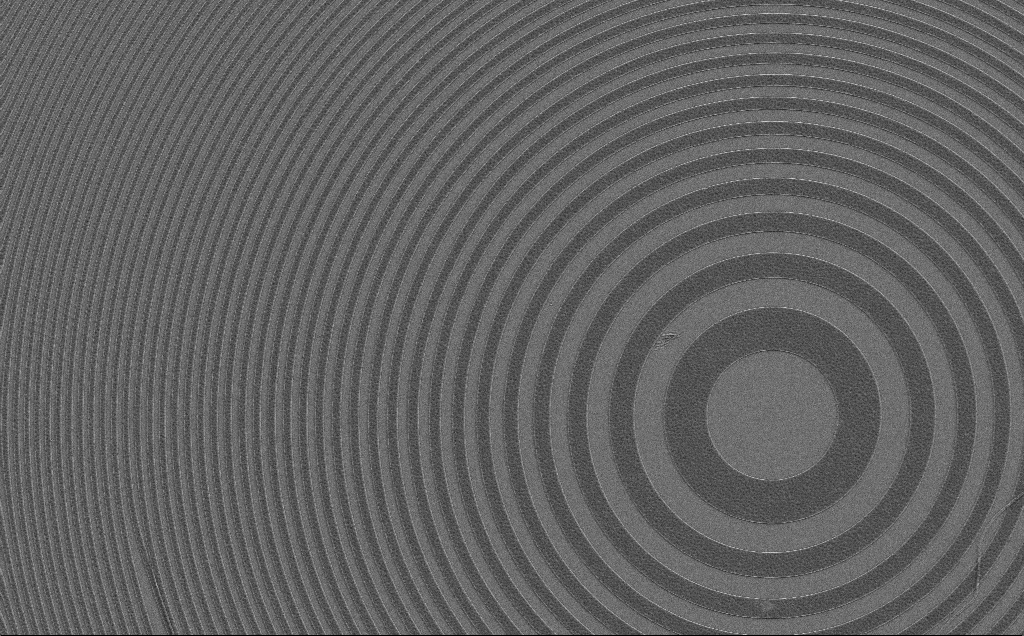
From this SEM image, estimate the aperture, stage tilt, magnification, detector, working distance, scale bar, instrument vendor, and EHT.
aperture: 30 µm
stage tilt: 0°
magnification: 1.61 K X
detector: SE2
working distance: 7 mm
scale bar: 10000 nm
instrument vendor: Zeiss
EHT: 5 kV